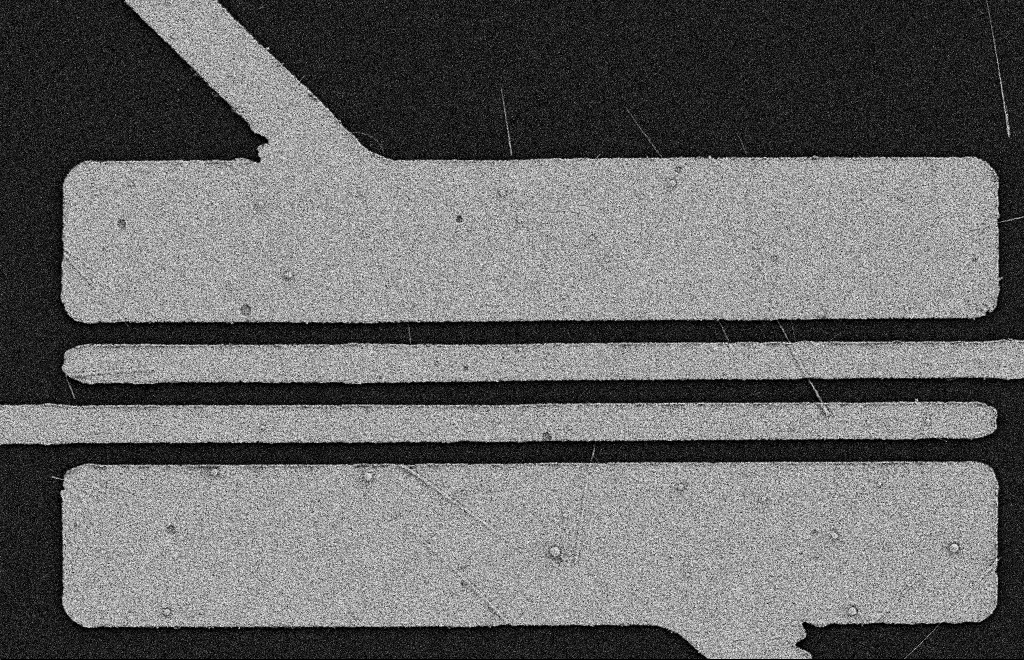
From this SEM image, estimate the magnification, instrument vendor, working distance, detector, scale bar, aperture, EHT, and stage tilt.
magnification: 5.56 K X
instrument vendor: Zeiss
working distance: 11 mm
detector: SE2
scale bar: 2000 nm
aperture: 20 µm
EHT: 2 kV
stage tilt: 0°